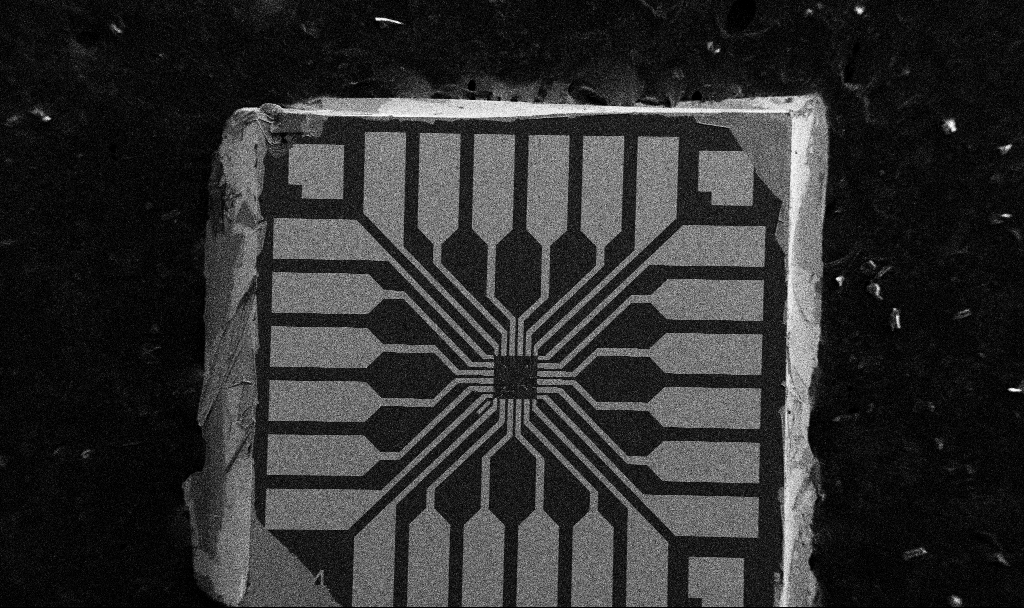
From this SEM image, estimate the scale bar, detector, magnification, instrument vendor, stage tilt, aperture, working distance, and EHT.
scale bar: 200000 nm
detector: SE2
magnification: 0.1 K X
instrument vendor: Zeiss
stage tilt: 0°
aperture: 30 µm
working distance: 10.7 mm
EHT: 5 kV